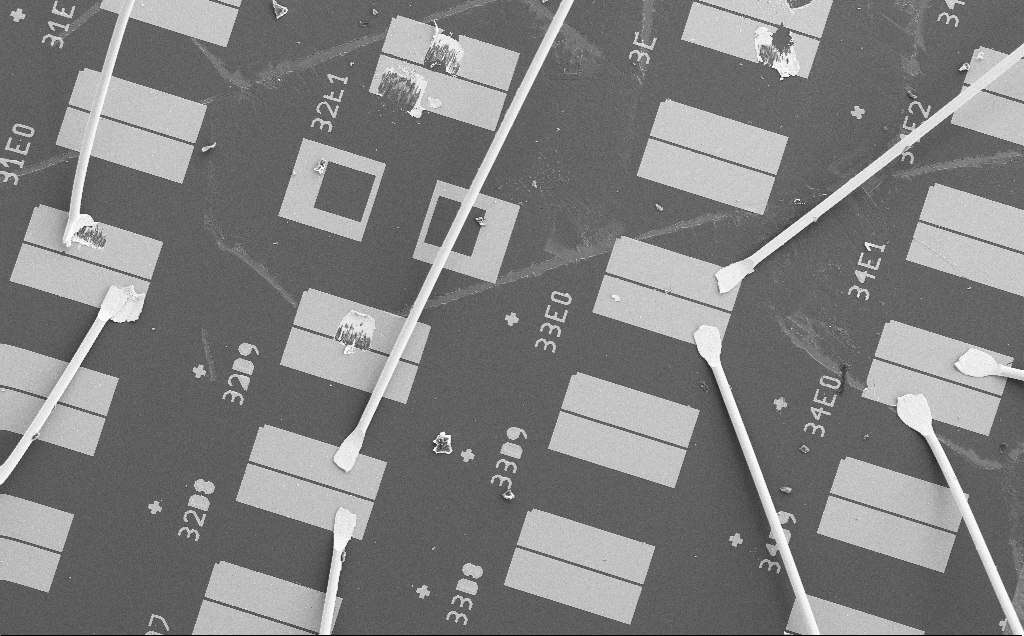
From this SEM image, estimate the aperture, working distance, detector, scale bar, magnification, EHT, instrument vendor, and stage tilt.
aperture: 30 µm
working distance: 10 mm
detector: SE2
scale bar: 200000 nm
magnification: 0.154 K X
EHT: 15 kV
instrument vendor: Zeiss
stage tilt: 0°